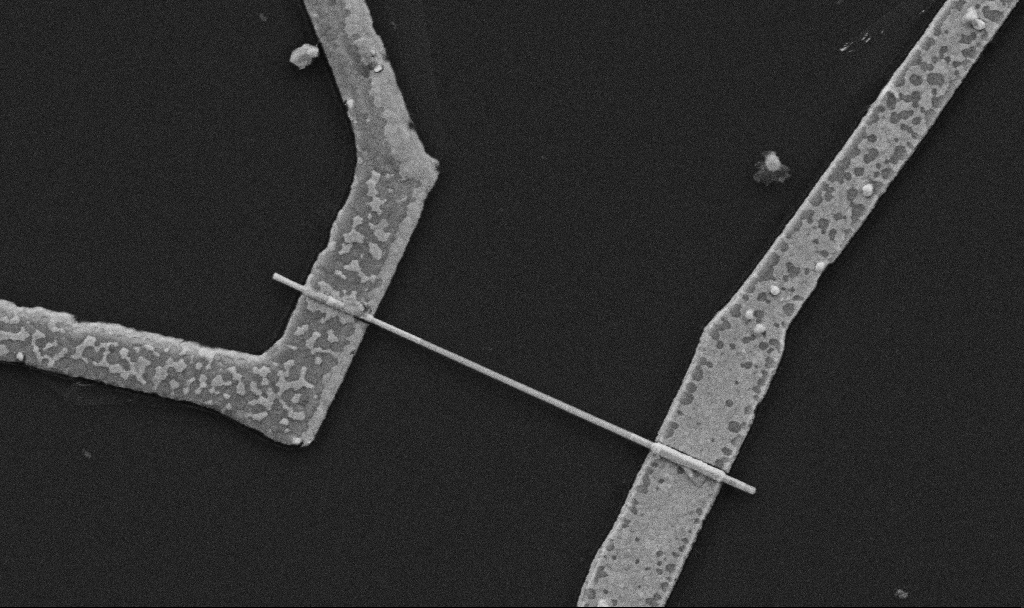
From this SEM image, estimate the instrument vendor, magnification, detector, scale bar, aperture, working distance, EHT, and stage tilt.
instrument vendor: Zeiss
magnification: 30 K X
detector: SE2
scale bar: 1000 nm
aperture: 30 µm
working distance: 10.7 mm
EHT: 5 kV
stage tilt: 0°